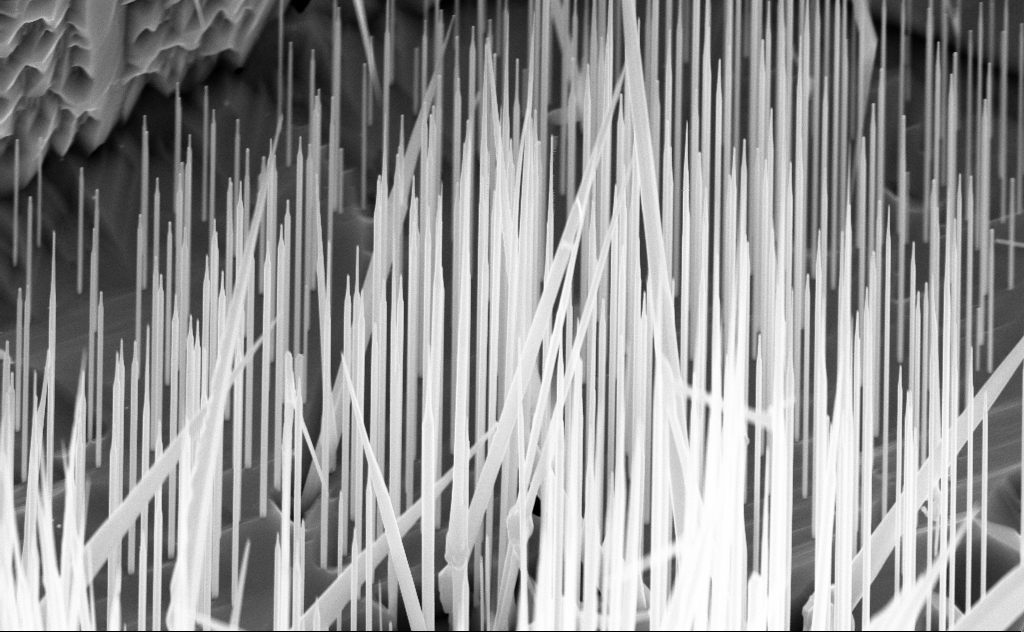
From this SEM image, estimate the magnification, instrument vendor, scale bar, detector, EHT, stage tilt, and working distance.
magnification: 25.94 K X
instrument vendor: Zeiss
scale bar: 2000 nm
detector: InLens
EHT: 10 kV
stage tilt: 45°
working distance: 6 mm